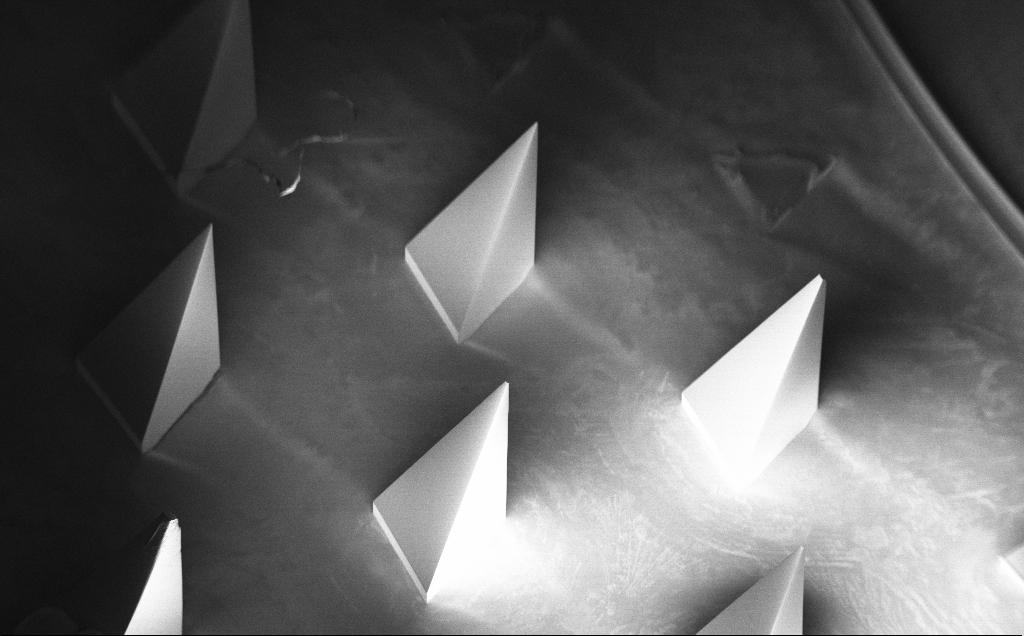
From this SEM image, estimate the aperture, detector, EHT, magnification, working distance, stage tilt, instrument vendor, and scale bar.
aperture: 30 µm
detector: InLens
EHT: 5 kV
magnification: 0.081 K X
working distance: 8 mm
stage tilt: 40°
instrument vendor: Zeiss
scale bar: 200000 nm